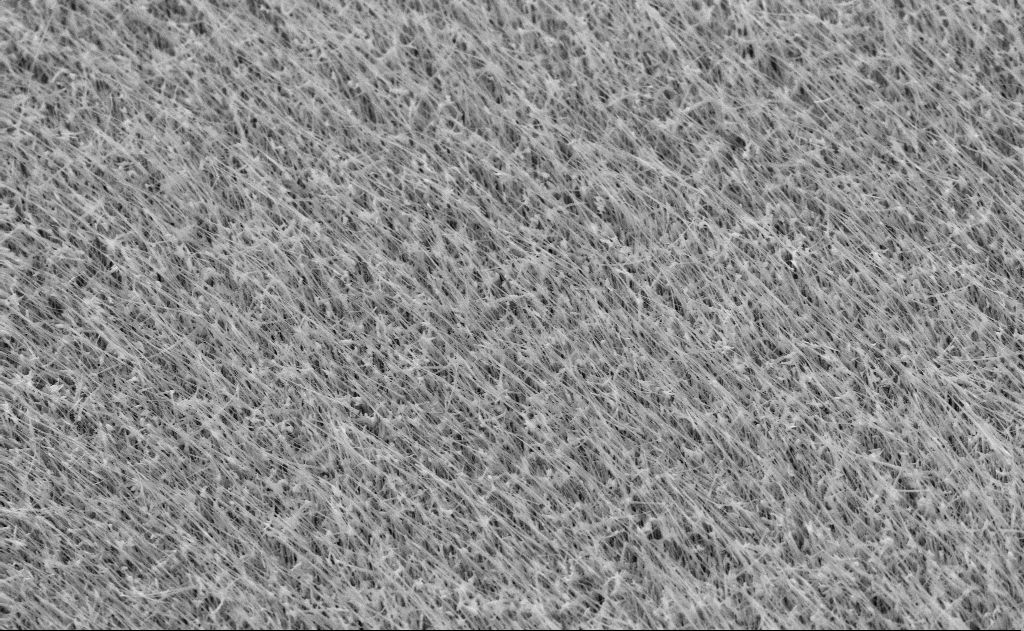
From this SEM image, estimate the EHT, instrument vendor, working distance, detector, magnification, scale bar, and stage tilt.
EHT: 10 kV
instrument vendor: Zeiss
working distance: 12 mm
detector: SE2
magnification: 10 K X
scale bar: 2000 nm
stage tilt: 45°